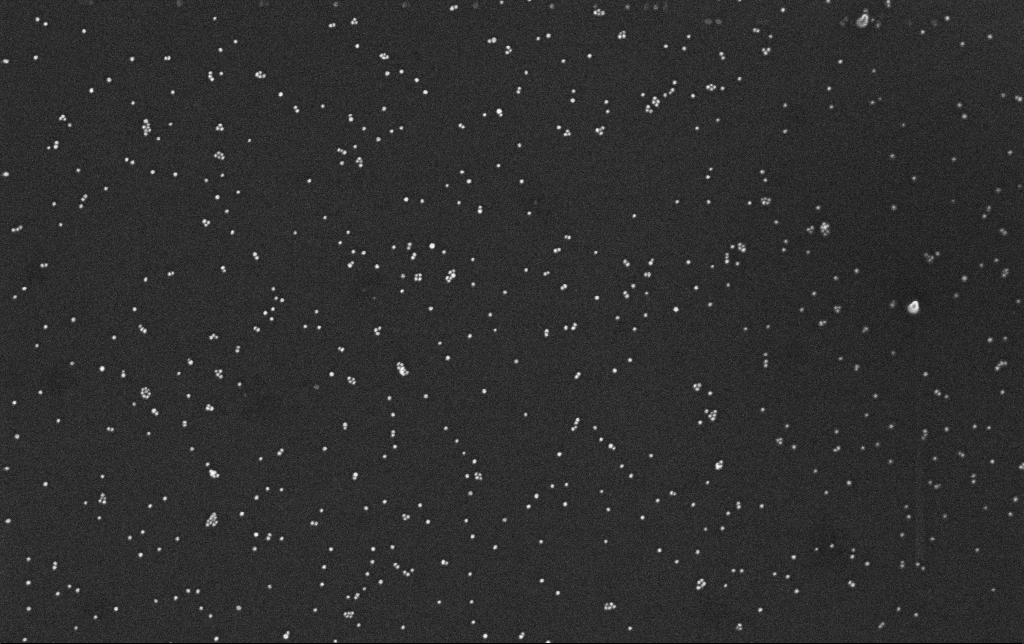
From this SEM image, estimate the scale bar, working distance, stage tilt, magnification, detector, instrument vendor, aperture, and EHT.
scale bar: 200 nm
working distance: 3.4 mm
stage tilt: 0°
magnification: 100 K X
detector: InLens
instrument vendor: Zeiss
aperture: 30 µm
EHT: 10 kV